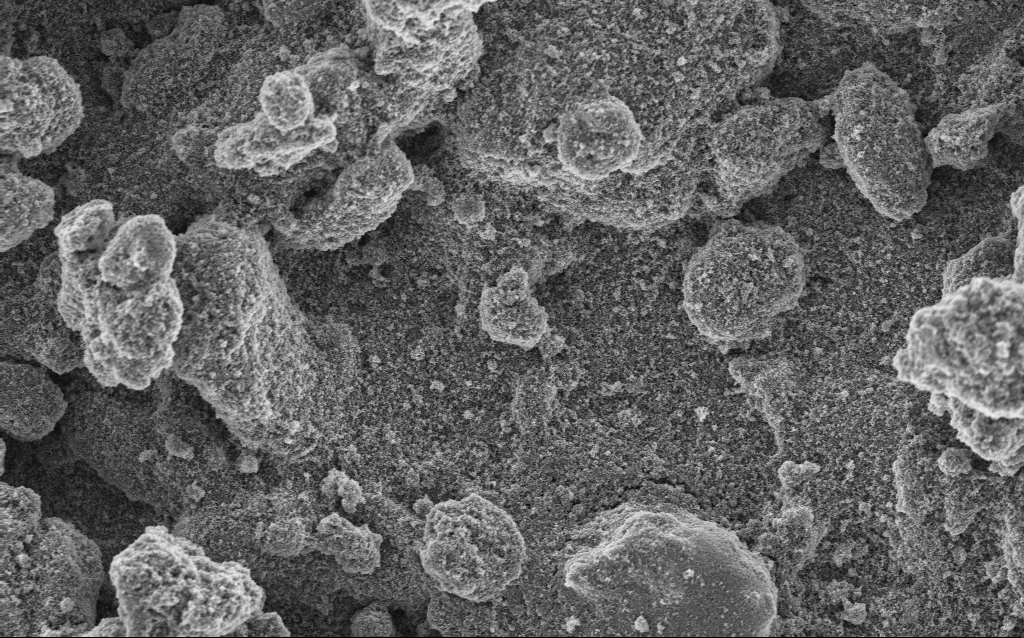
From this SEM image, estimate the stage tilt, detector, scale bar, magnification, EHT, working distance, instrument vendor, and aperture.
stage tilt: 0°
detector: InLens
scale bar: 10000 nm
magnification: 6.41 K X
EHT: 5 kV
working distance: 4.2 mm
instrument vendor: Zeiss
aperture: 30 µm